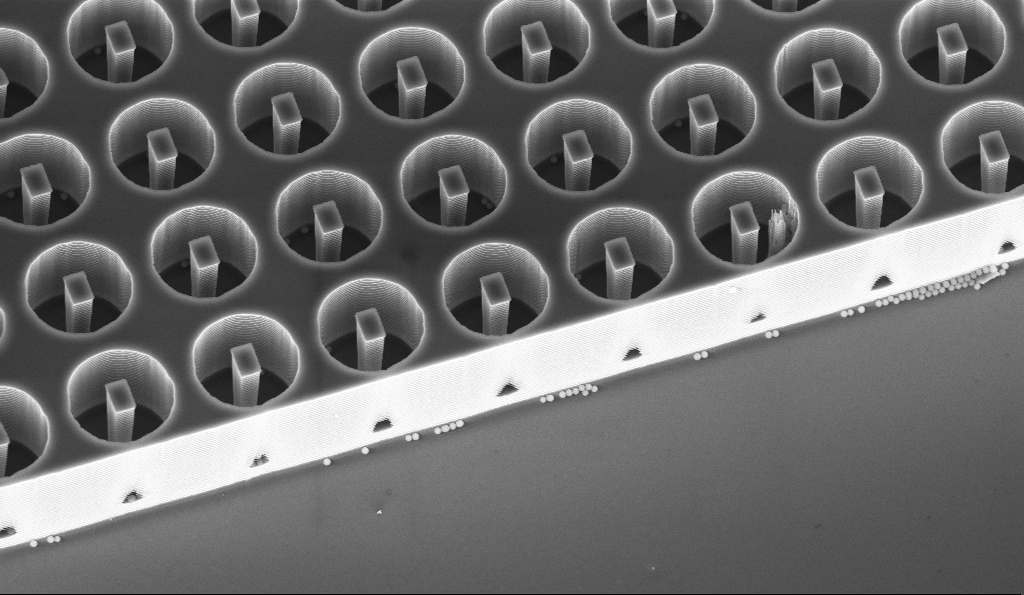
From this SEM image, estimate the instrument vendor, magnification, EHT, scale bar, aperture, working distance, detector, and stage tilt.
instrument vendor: Zeiss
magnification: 4.3 K X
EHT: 10 kV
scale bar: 10000 nm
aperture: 30 µm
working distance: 14.1 mm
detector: InLens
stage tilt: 30°